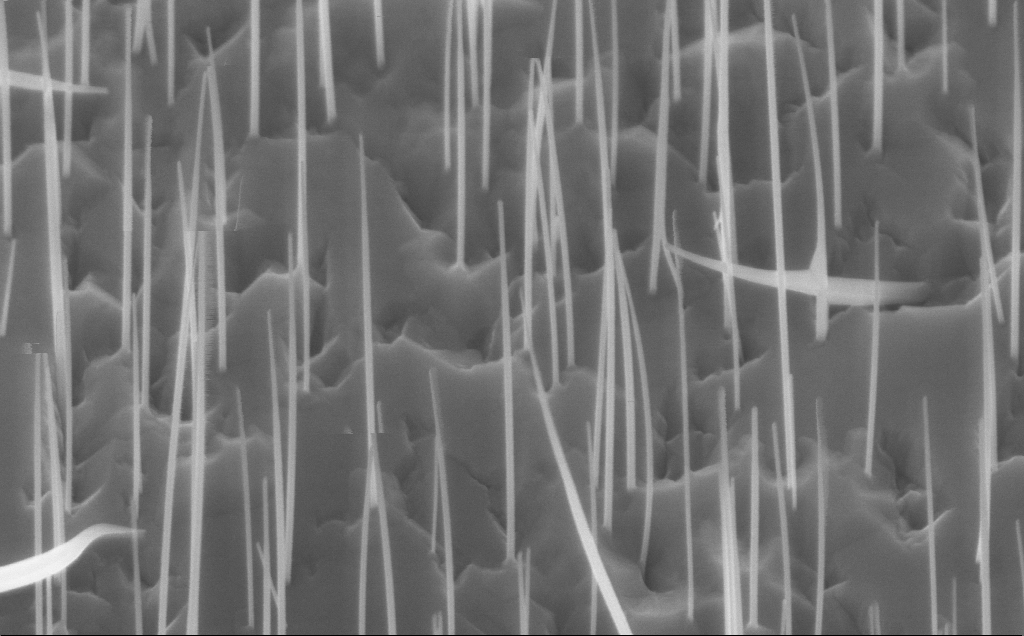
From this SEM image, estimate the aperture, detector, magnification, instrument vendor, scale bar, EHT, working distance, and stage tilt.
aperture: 30 µm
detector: InLens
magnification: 80 K X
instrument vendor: Zeiss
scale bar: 200 nm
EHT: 10 kV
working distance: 7 mm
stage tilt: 45°